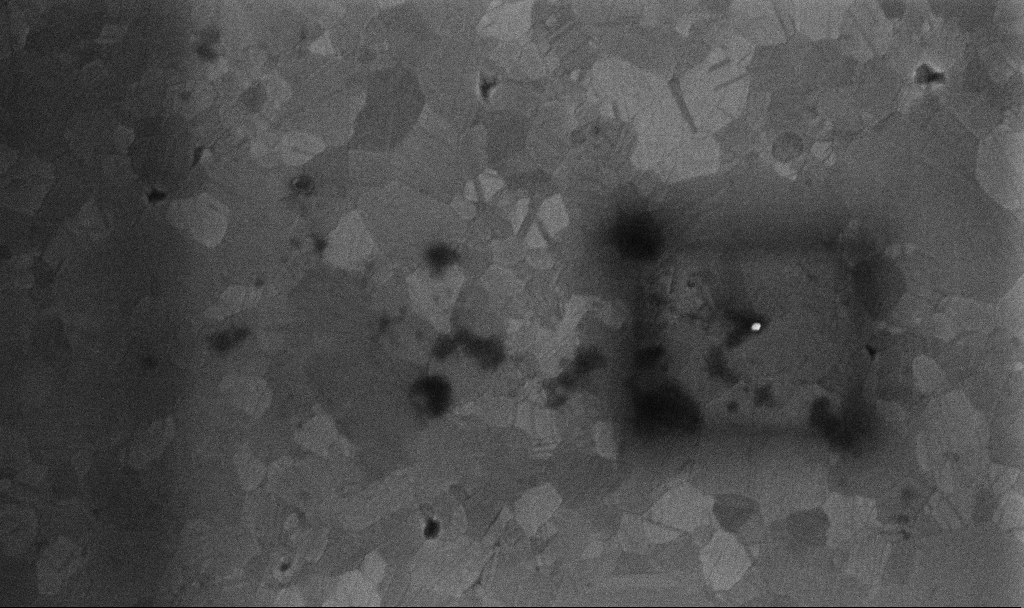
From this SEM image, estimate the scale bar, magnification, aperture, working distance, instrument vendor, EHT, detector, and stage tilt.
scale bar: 200 nm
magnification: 99.87 K X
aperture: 30 µm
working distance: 3.3 mm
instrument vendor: Zeiss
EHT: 10 kV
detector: InLens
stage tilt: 0°